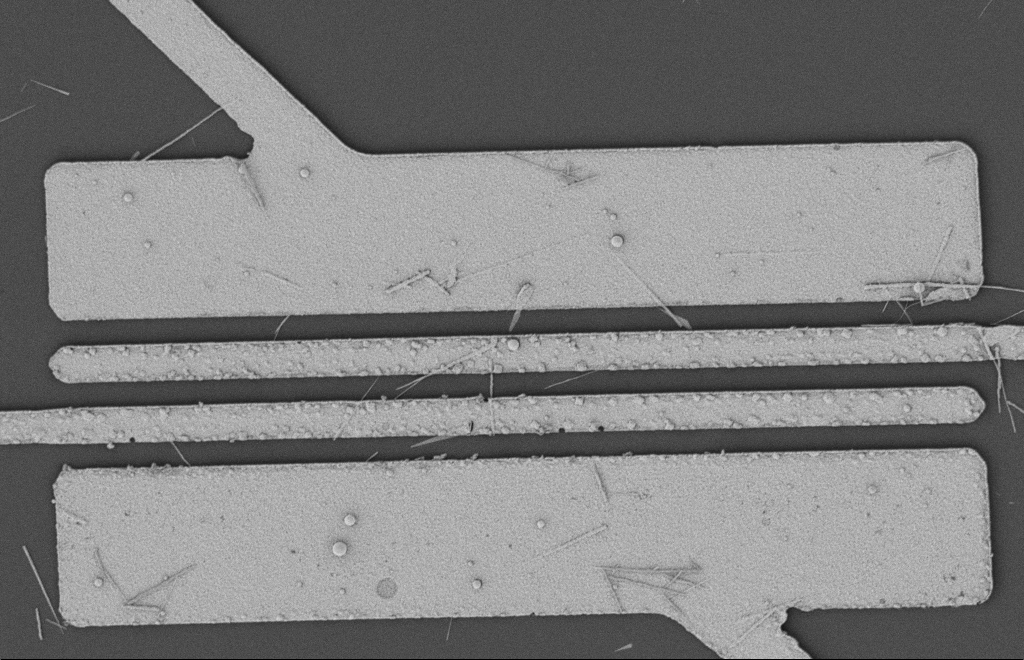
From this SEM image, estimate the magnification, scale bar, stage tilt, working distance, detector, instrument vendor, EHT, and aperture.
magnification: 5.59 K X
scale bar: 2000 nm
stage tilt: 0°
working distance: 12 mm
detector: SE2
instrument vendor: Zeiss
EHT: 2 kV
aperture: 20 µm